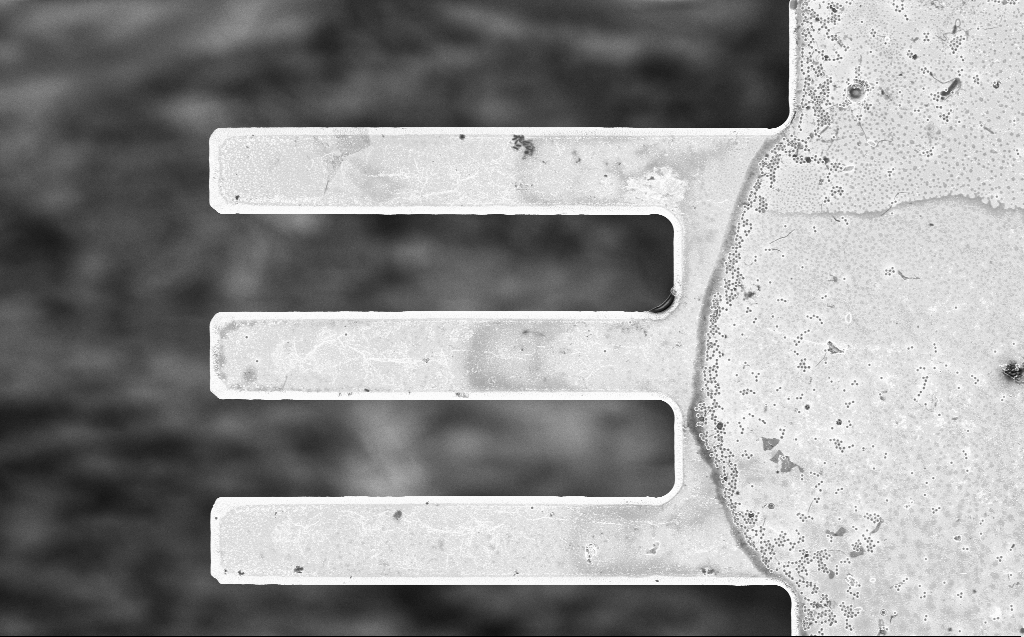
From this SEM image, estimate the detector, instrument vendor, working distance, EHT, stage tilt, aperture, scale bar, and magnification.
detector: InLens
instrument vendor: Zeiss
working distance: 7 mm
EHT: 3 kV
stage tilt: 0°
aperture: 30 µm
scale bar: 20000 nm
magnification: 1.71 K X